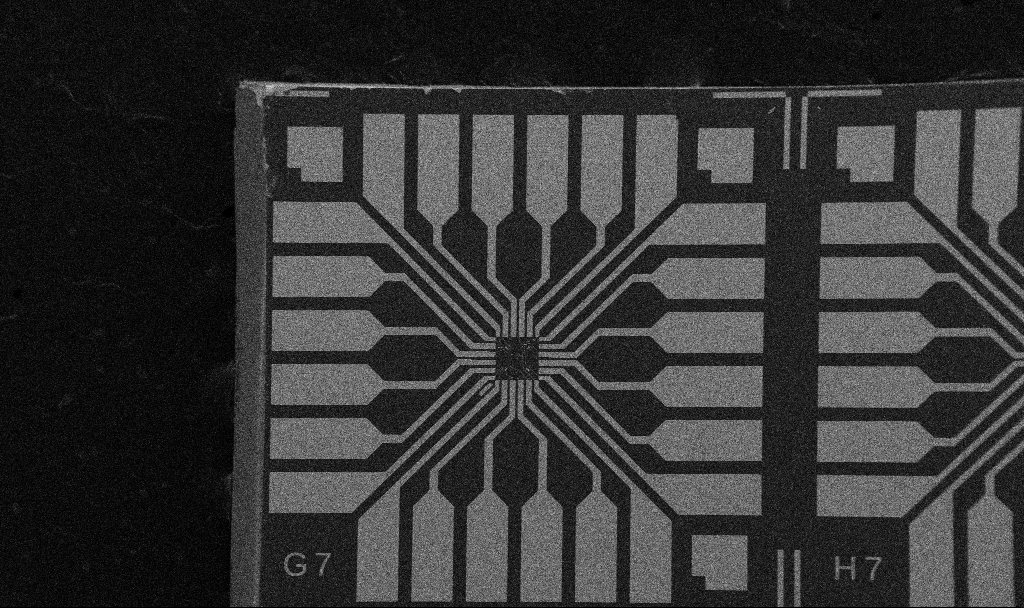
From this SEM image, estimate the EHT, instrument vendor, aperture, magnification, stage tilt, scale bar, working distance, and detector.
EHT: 5 kV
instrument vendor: Zeiss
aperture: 30 µm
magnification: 0.1 K X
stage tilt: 0°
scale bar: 200000 nm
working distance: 10.7 mm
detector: SE2